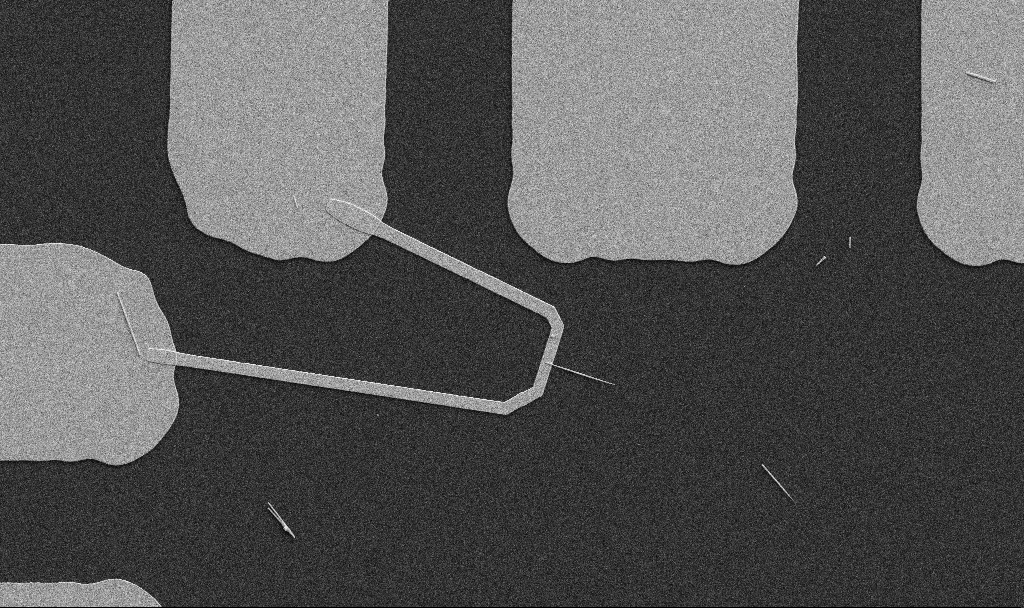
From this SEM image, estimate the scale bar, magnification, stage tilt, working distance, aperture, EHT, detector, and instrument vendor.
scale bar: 10000 nm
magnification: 5 K X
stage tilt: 0°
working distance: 10.7 mm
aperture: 30 µm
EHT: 5 kV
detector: SE2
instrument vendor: Zeiss